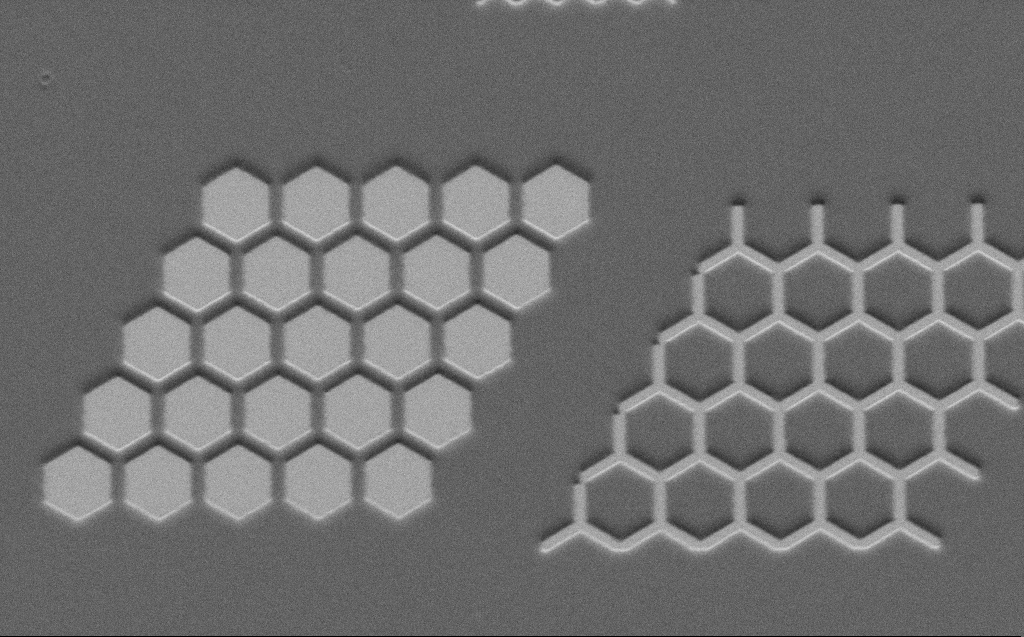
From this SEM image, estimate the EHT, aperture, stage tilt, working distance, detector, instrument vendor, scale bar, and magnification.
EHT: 3 kV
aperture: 30 µm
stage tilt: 45°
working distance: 6 mm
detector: SE2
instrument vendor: Zeiss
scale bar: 10000 nm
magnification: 4.74 K X